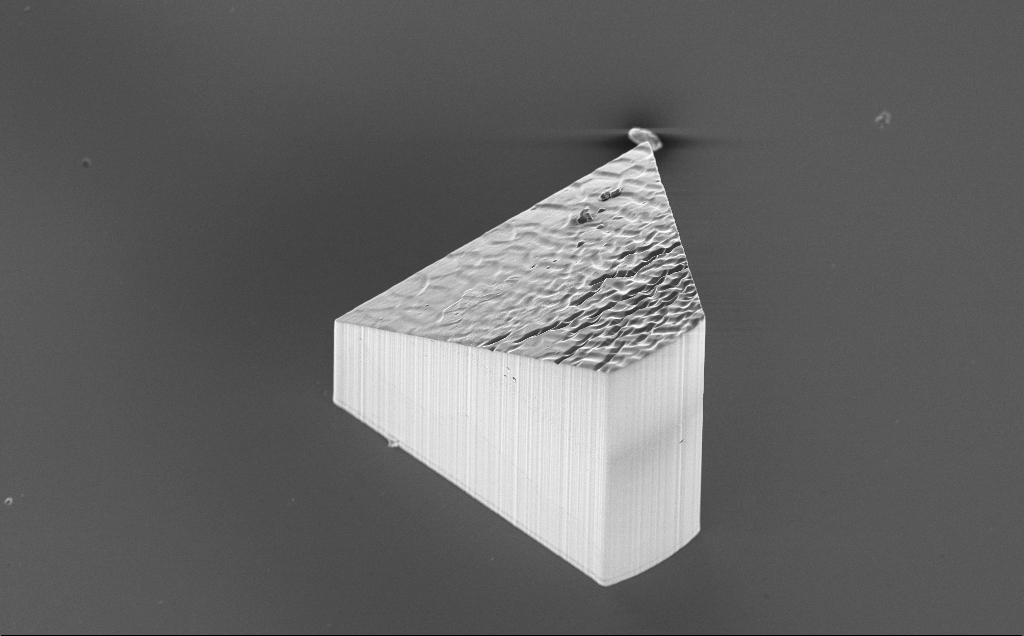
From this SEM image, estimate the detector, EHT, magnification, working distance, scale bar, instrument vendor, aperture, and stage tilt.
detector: InLens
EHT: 10 kV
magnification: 0.344 K X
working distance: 9 mm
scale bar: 100000 nm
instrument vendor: Zeiss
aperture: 30 µm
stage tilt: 20°